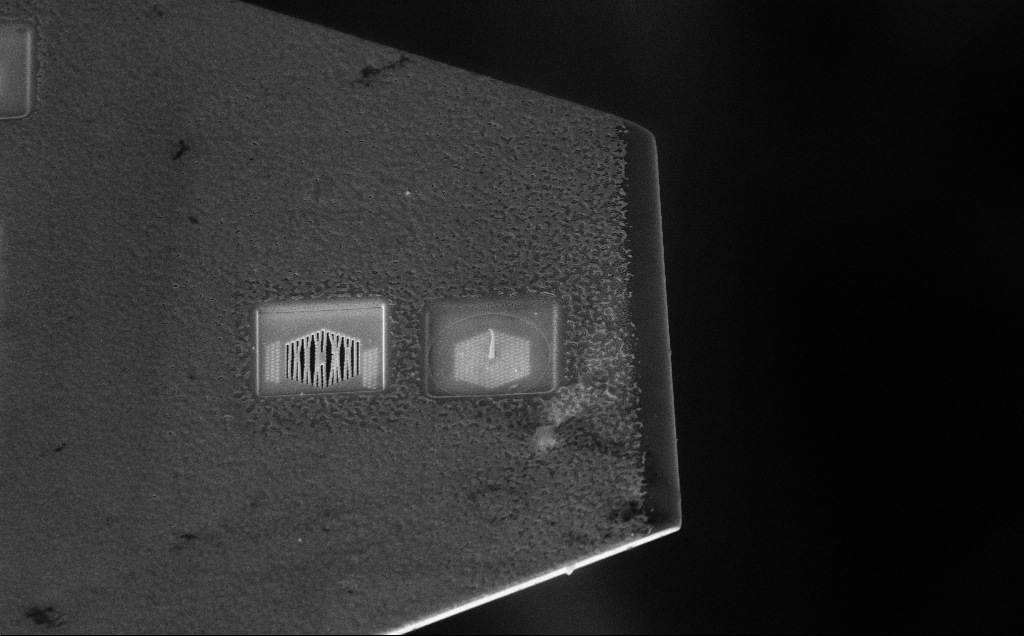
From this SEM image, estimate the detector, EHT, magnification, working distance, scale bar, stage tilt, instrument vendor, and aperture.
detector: InLens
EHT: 10 kV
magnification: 7.38 K X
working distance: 11 mm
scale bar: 2000 nm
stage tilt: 45°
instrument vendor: Zeiss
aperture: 30 µm